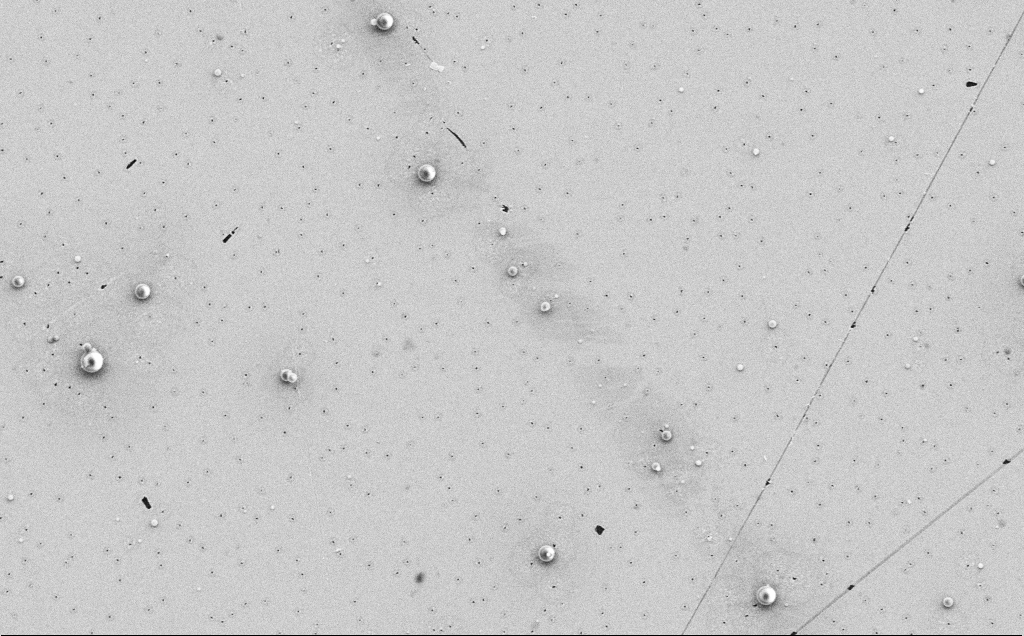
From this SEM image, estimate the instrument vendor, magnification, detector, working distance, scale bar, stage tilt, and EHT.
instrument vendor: Zeiss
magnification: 4.2 K X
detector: SE2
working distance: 12 mm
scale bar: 10000 nm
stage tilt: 0°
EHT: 5 kV